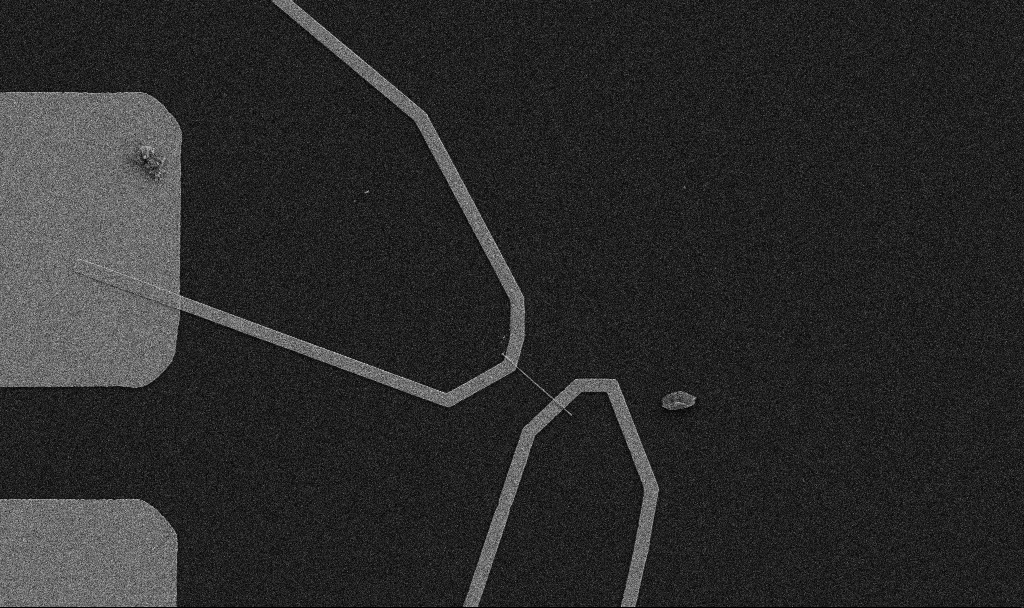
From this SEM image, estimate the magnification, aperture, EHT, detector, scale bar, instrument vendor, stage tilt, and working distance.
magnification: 5 K X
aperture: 30 µm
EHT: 5 kV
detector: SE2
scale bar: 10000 nm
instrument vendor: Zeiss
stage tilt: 0°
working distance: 10.7 mm